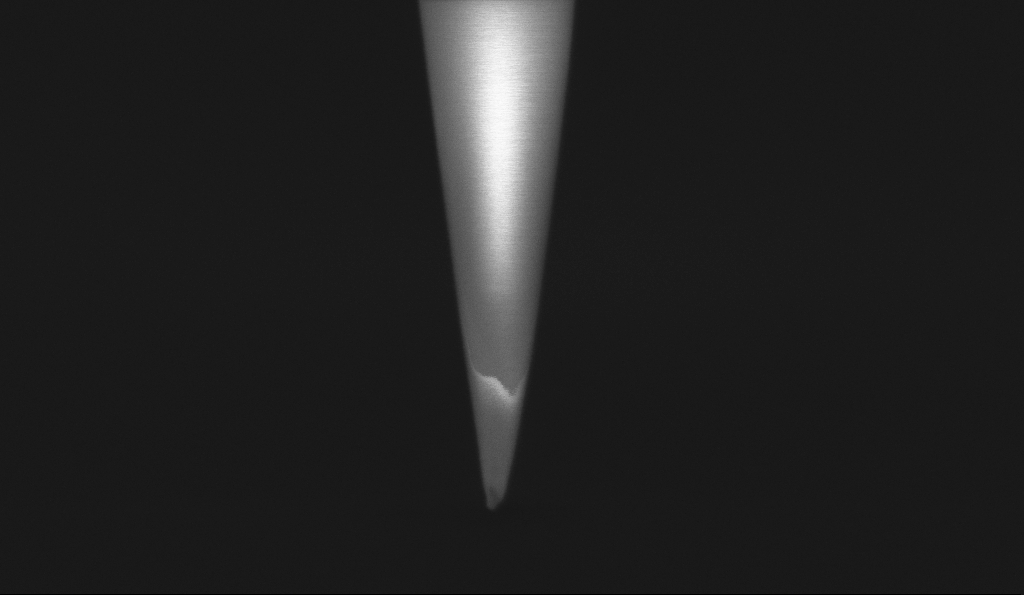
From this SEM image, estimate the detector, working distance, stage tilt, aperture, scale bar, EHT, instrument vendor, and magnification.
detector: InLens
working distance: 5.6 mm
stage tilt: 0°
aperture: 30 µm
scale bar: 200 nm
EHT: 1 kV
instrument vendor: Zeiss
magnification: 100 K X